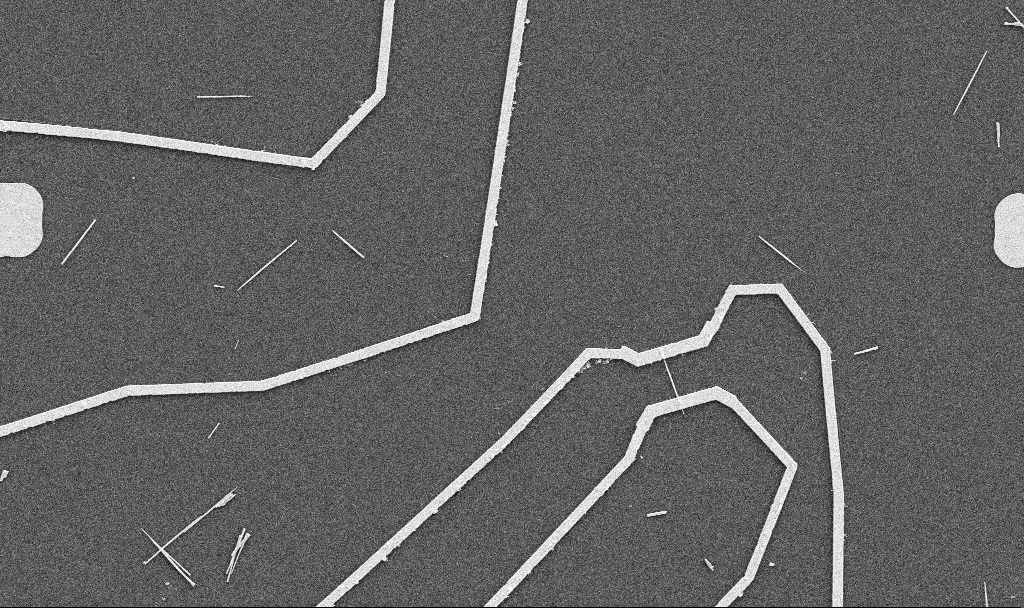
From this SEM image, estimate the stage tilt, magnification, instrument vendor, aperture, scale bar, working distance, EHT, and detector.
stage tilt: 0°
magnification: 5 K X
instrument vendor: Zeiss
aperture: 30 µm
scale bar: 10000 nm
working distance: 10.7 mm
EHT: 5 kV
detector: SE2